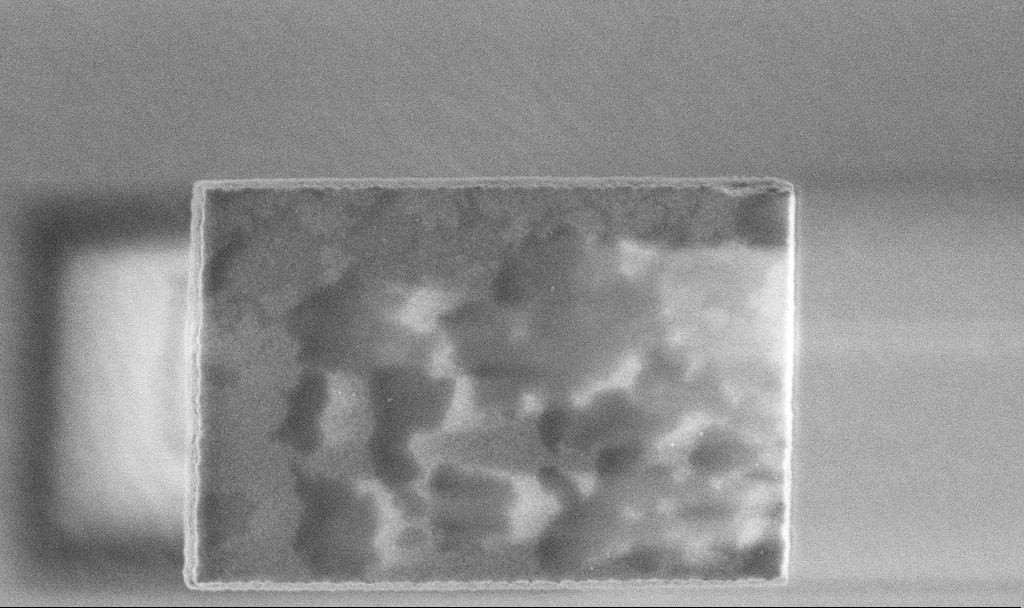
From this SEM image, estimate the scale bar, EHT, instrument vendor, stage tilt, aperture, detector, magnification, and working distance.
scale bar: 1000 nm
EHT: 3 kV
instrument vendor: Zeiss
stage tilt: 0°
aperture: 30 µm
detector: InLens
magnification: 49.65 K X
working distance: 3.3 mm